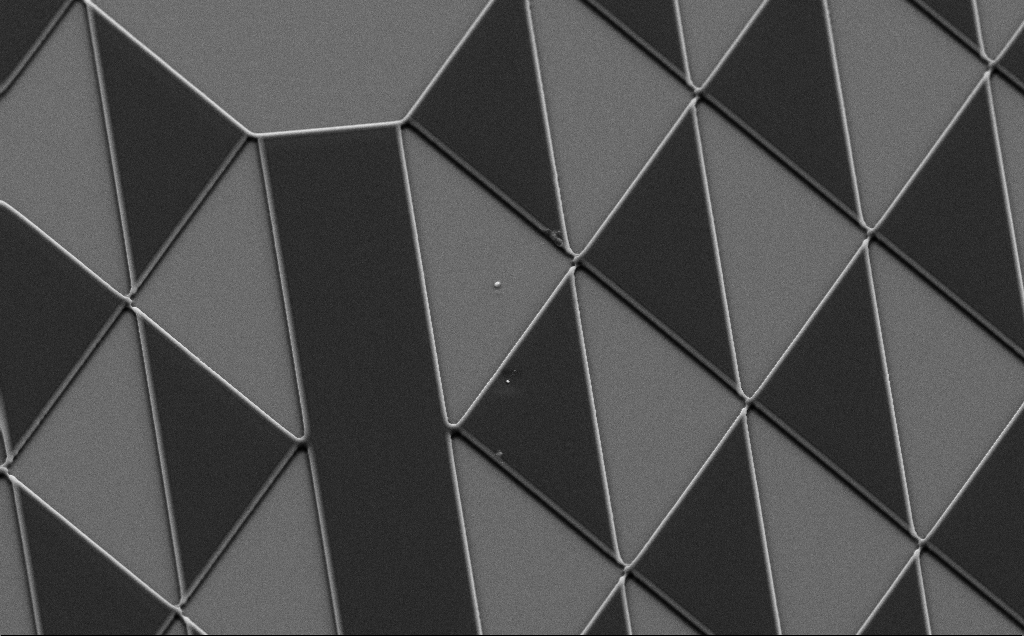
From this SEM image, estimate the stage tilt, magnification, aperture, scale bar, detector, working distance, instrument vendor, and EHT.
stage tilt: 35°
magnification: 1.55 K X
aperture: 30 µm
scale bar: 10000 nm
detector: SE2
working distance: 8 mm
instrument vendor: Zeiss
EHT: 10 kV